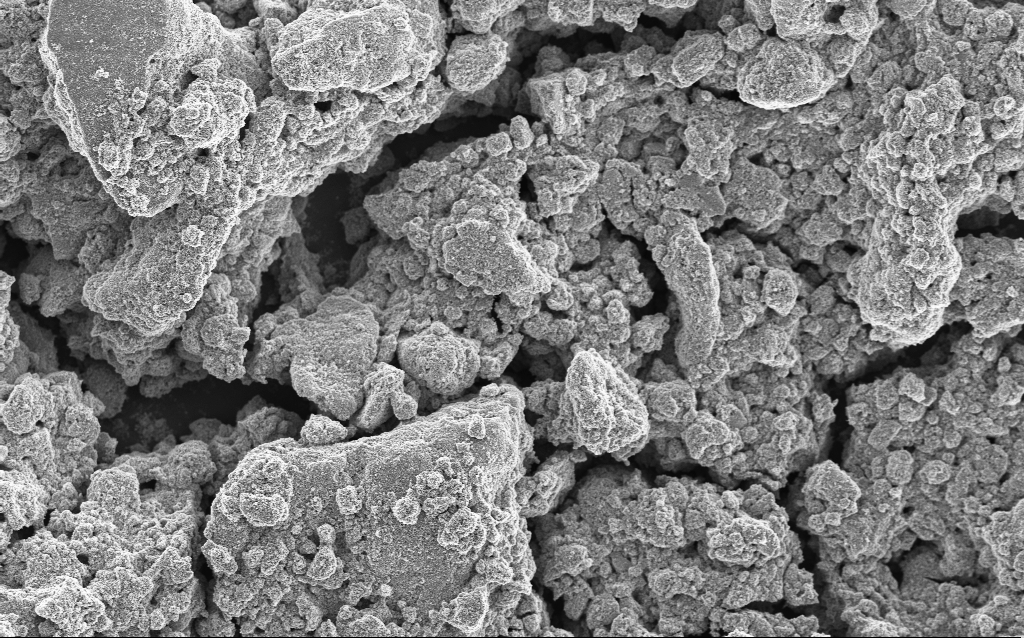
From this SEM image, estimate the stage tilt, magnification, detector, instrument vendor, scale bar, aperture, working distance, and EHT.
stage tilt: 0°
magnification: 1.23 K X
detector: InLens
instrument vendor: Zeiss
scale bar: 10000 nm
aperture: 30 µm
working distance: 4.5 mm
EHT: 5 kV